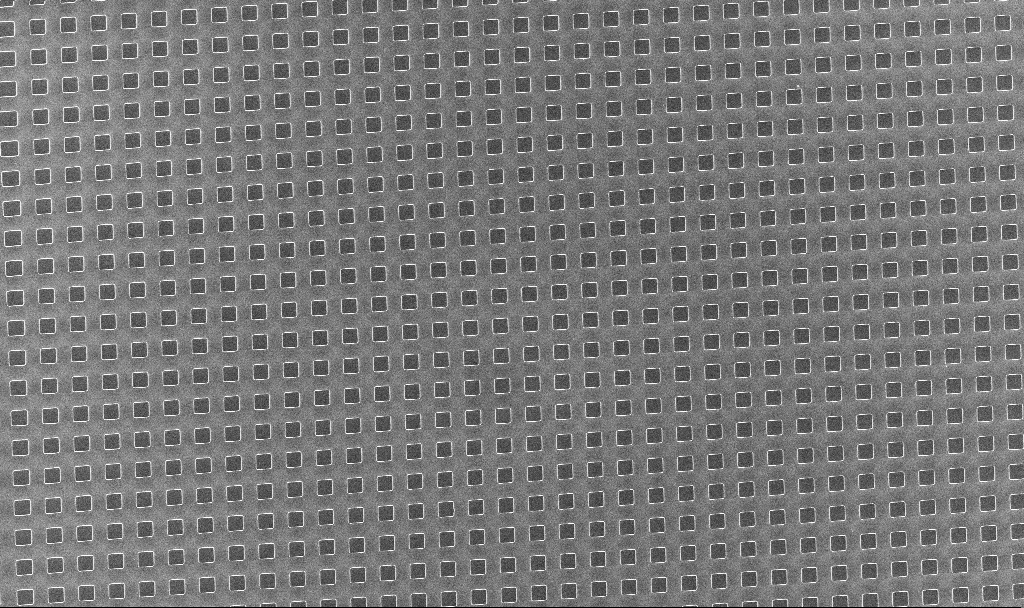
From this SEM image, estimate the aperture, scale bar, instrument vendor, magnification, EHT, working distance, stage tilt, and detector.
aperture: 30 µm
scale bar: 100000 nm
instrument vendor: Zeiss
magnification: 0.692 K X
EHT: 5 kV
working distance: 5.3 mm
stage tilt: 0°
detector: InLens